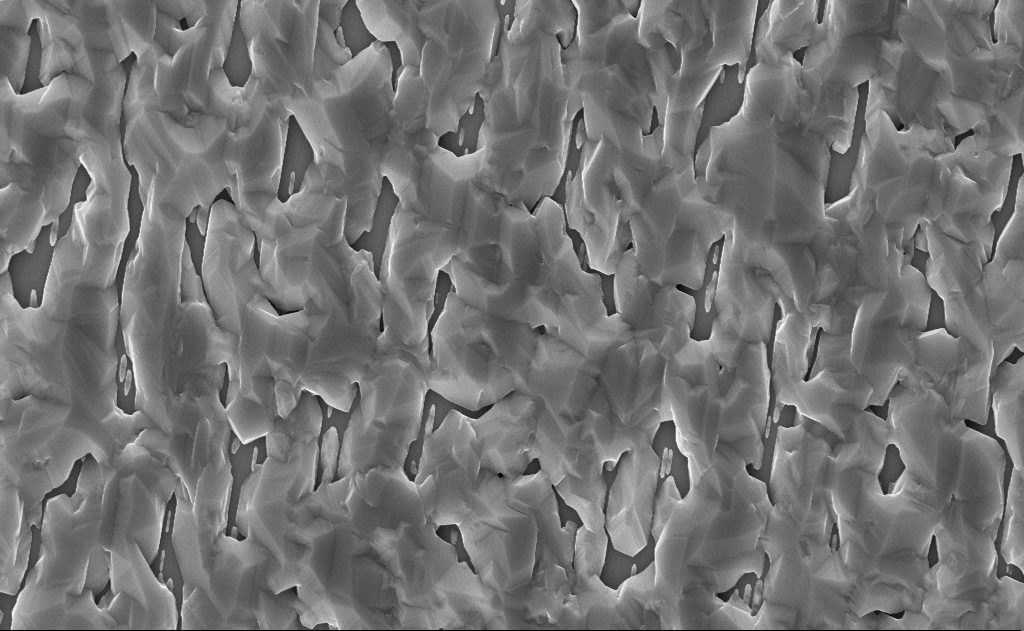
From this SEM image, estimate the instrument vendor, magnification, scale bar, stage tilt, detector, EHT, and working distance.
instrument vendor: Zeiss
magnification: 20 K X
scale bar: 2000 nm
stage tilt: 0°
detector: InLens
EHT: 10 kV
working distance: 14 mm